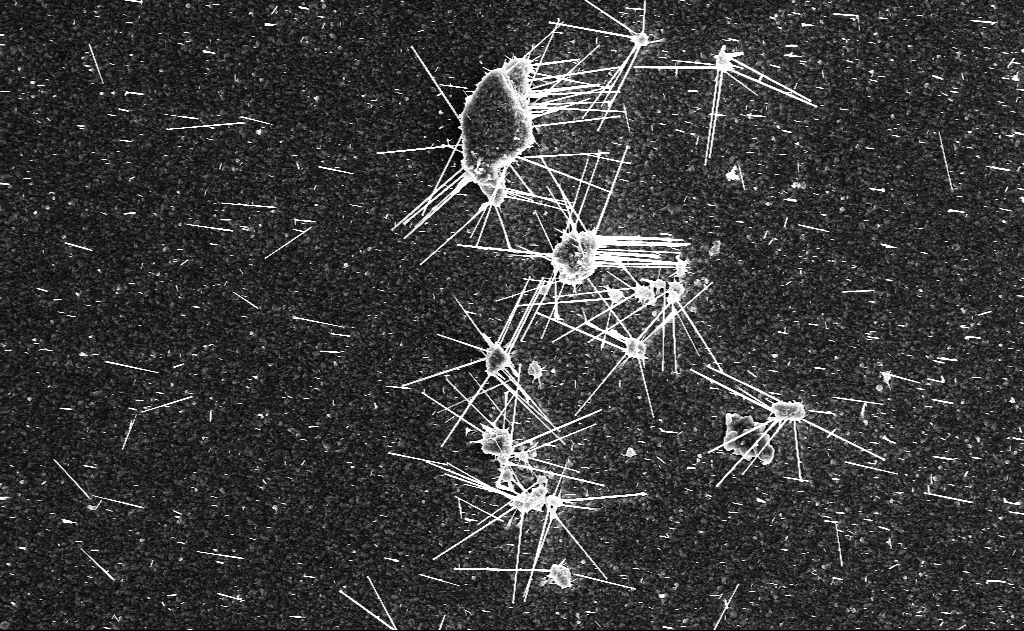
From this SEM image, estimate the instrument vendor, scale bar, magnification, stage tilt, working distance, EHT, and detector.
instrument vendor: Zeiss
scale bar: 10000 nm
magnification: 5 K X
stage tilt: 0°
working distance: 9 mm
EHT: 10 kV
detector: InLens